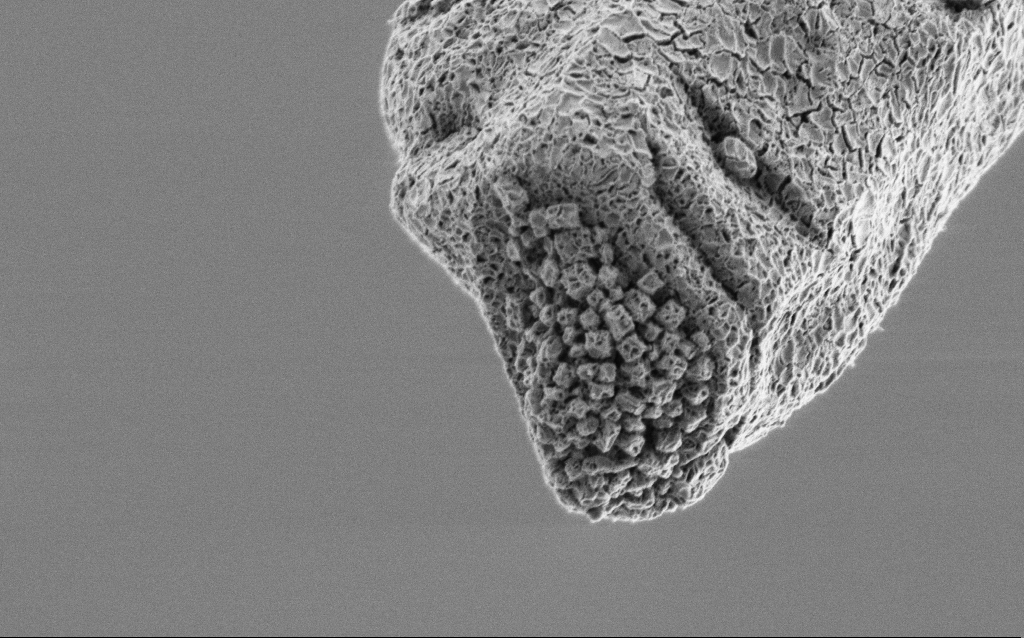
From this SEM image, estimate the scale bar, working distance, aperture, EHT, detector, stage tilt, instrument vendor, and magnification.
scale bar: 2000 nm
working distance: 6.8 mm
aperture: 30 µm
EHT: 1 kV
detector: SE2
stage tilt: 45°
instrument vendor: Zeiss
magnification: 25 K X